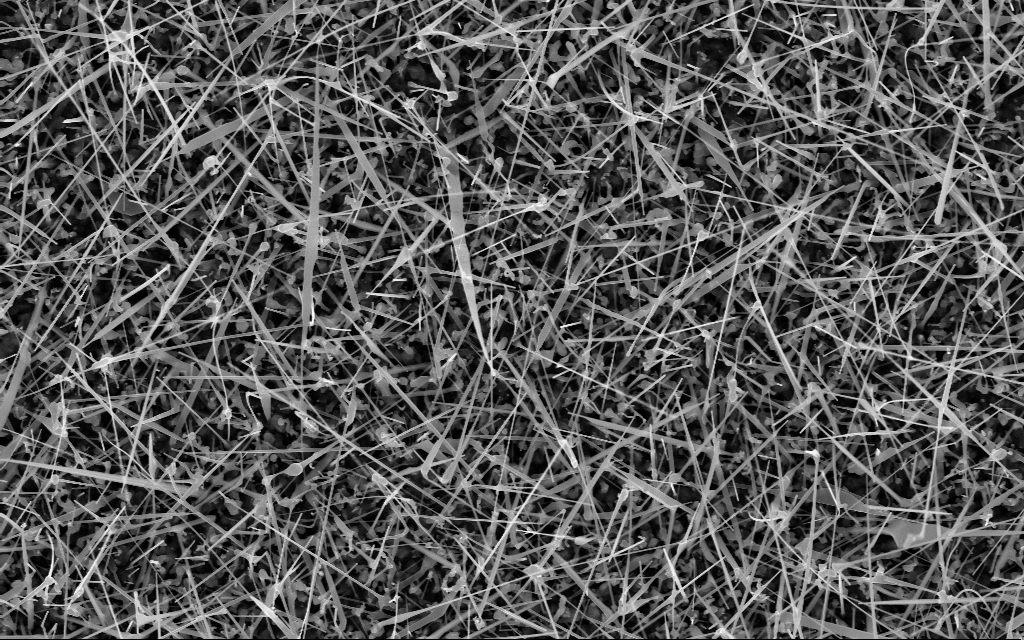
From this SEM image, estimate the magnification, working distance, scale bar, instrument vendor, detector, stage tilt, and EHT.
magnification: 20 K X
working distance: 7 mm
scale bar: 2000 nm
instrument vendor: Zeiss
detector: InLens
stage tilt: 0°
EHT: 10 kV